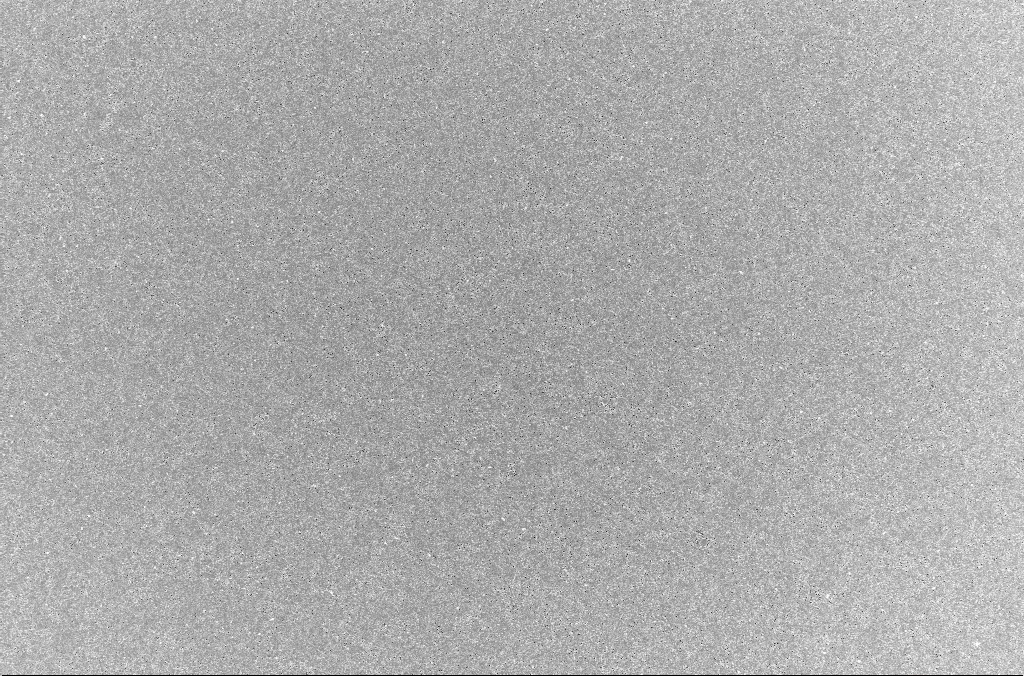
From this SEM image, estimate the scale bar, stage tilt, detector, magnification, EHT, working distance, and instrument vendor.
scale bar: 20000 nm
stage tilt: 0°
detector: InLens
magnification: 1 K X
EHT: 5 kV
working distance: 3 mm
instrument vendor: Zeiss